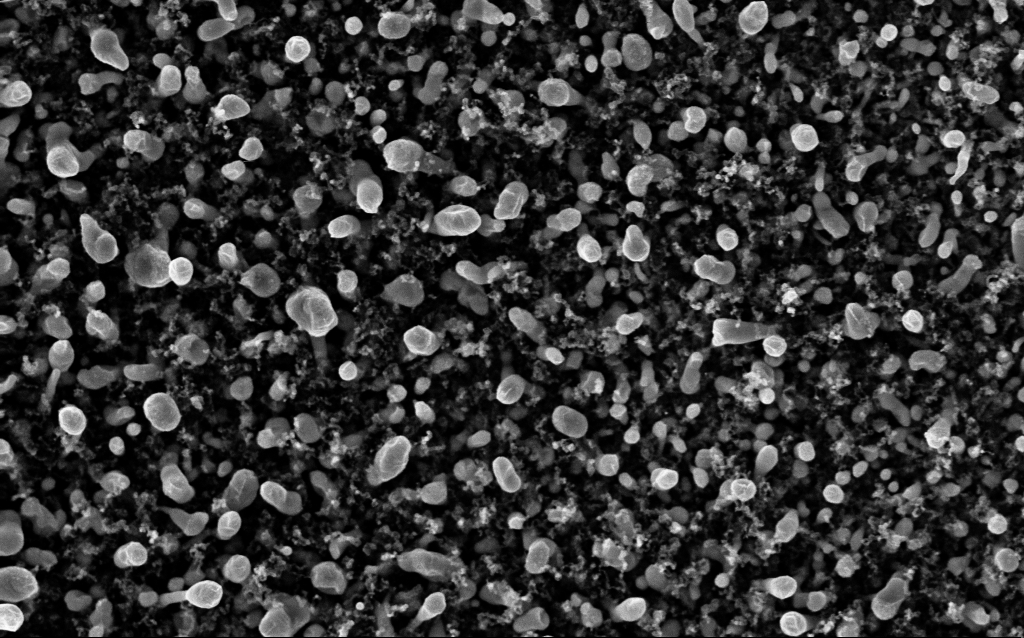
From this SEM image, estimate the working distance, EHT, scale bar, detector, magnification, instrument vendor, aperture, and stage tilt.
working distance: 2.3 mm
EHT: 5 kV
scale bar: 1000 nm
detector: InLens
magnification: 50 K X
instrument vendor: Zeiss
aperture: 30 µm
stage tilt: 0°